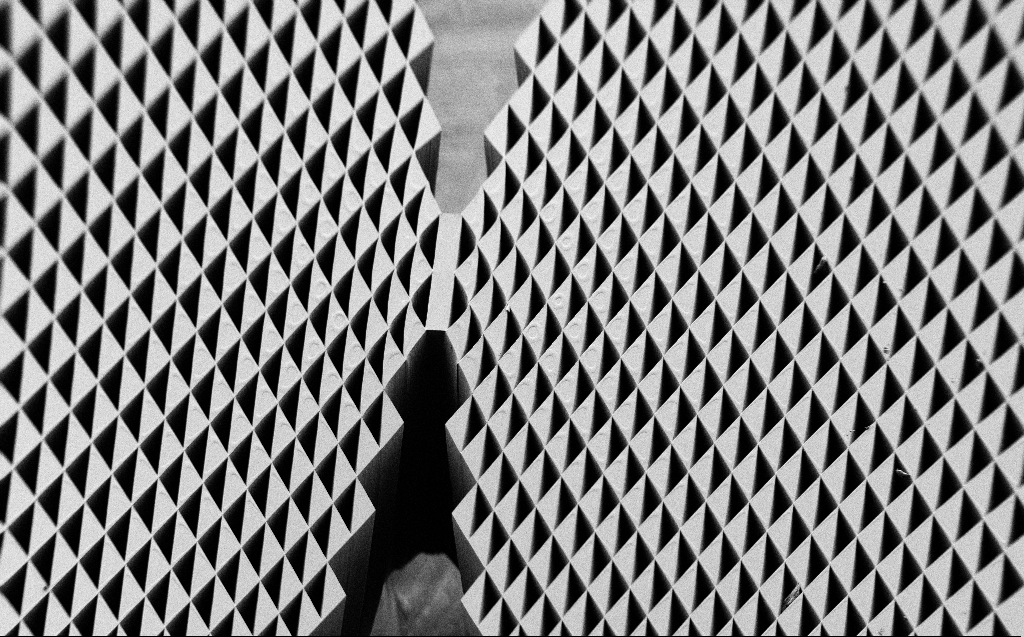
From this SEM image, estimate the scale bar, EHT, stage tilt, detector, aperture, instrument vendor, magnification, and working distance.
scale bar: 200000 nm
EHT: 1 kV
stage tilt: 45°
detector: SE2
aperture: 30 µm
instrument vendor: Zeiss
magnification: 0.224 K X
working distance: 5 mm